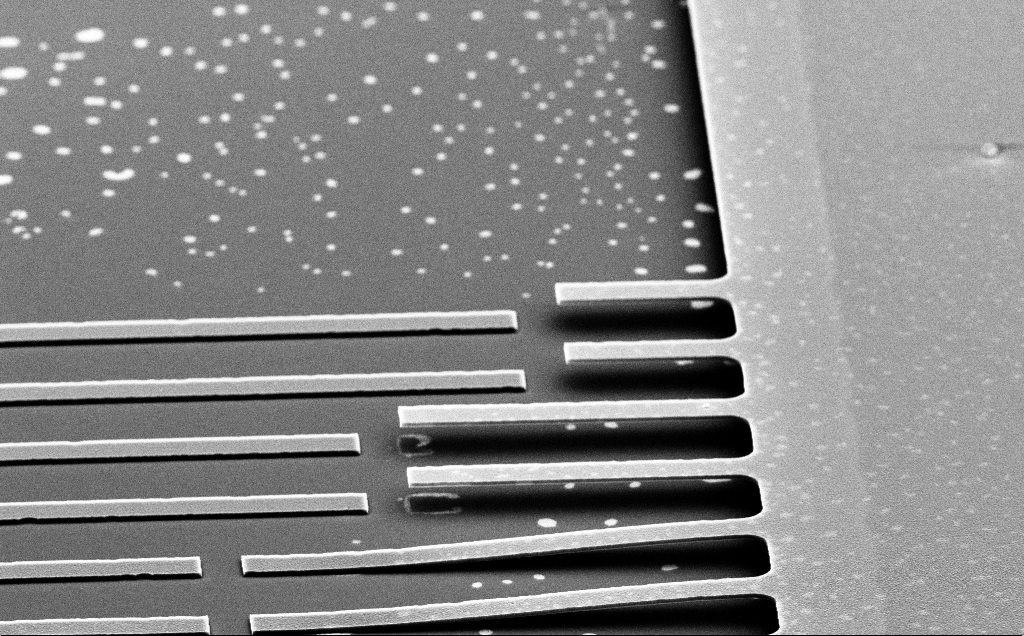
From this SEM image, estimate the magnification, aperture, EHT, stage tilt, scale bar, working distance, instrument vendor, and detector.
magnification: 2.56 K X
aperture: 30 µm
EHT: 10 kV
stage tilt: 65.4°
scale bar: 20000 nm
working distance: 18 mm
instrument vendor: Zeiss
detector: SE2